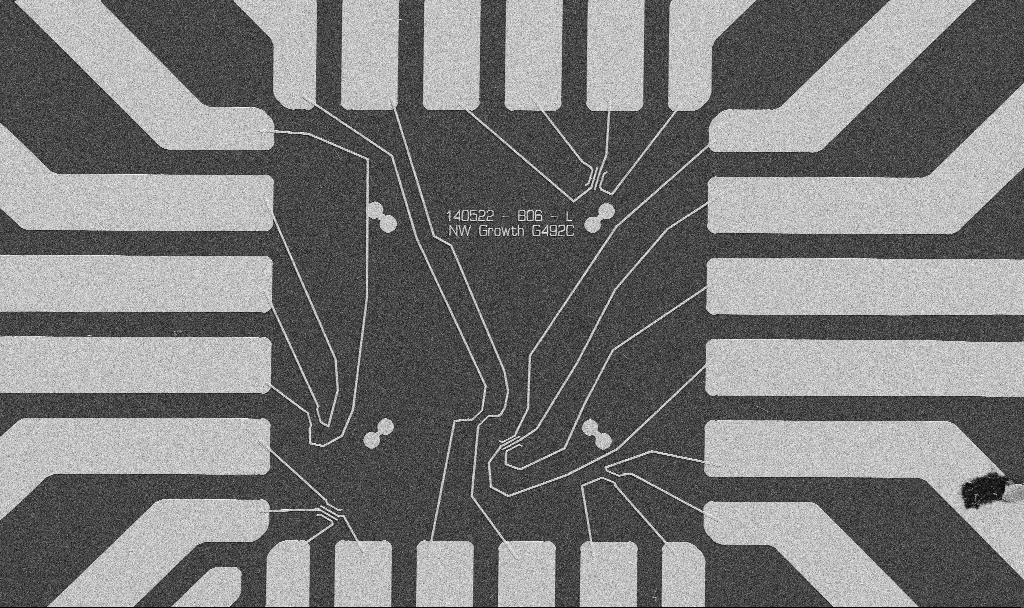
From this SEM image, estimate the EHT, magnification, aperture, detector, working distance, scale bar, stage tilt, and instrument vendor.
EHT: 5 kV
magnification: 1 K X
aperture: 30 µm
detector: SE2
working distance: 10.7 mm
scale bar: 20000 nm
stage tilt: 0°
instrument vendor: Zeiss